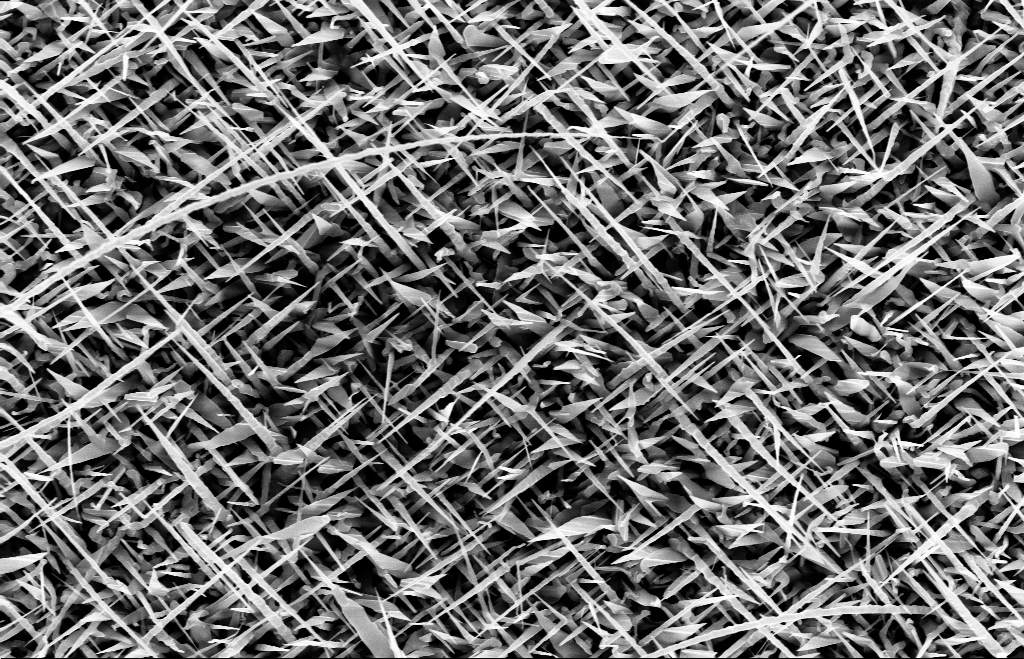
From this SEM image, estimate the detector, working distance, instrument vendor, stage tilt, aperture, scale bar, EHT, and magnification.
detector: InLens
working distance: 8 mm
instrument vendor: Zeiss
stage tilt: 0°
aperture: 30 µm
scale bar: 1000 nm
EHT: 10 kV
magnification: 20 K X